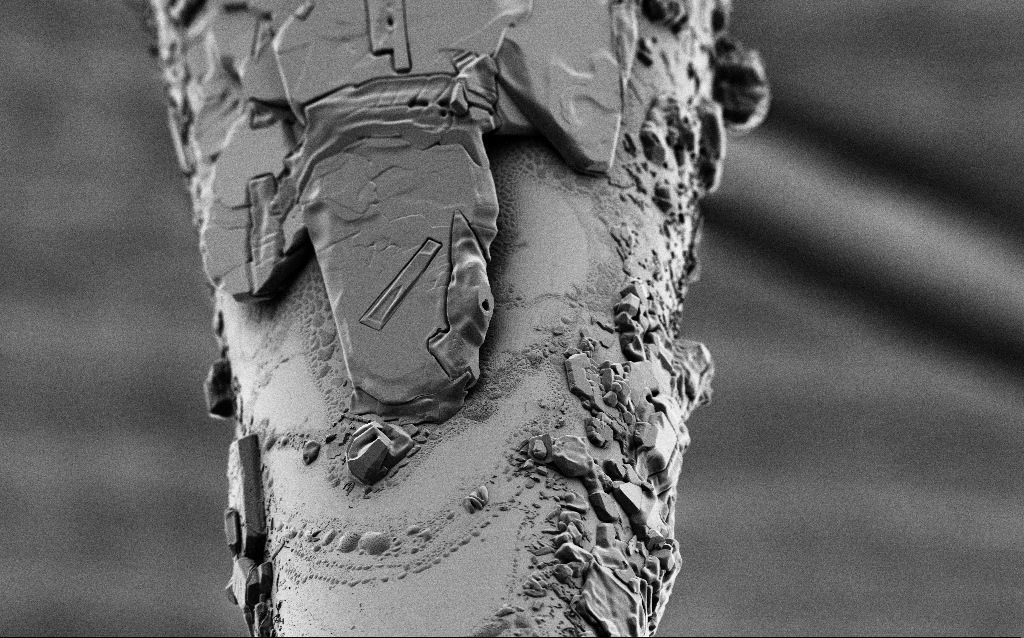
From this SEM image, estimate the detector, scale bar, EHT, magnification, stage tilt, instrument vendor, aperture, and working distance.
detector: SE2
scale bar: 20000 nm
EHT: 1 kV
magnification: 0.75 K X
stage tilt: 45°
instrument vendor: Zeiss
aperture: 30 µm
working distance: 6.4 mm